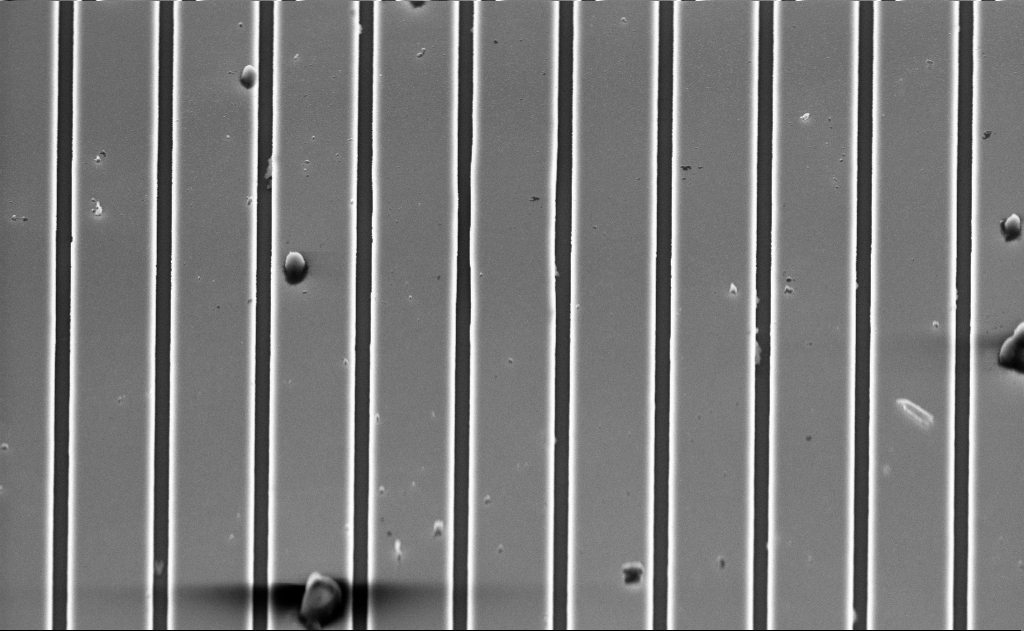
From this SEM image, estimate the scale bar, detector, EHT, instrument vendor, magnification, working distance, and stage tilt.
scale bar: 2000 nm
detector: InLens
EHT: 5 kV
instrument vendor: Zeiss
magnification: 9.22 K X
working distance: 5 mm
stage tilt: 45°